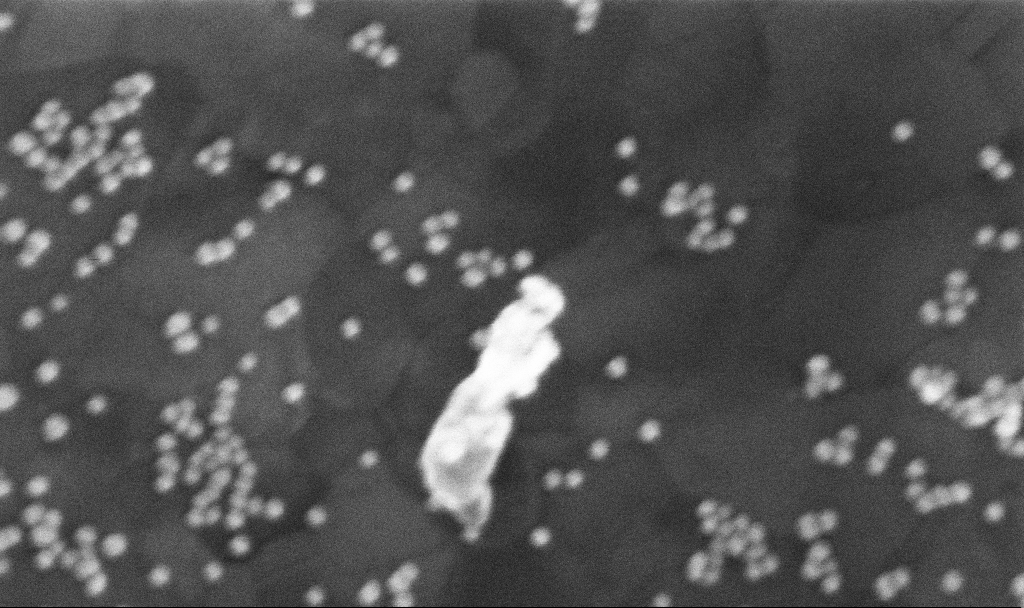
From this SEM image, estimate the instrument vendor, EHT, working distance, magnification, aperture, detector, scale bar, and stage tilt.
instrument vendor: Zeiss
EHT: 10 kV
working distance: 3.7 mm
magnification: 351.96 K X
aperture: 30 µm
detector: InLens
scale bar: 200 nm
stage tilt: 0°